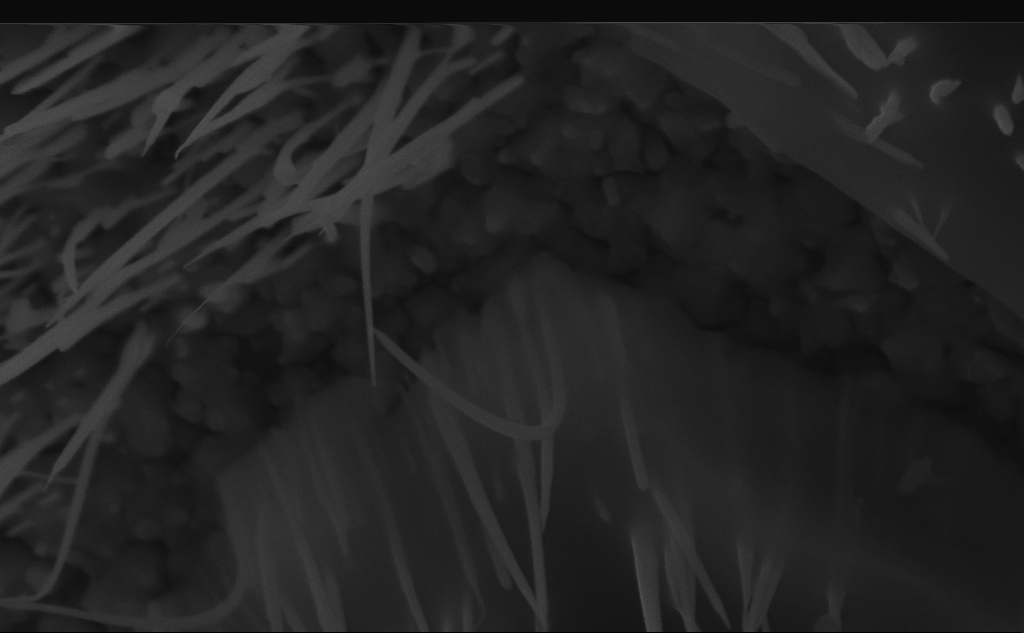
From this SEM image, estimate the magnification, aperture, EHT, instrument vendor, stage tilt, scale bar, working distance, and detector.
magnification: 98.96 K X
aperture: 30 µm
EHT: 10 kV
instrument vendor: Zeiss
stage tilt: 45°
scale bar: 200 nm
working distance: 6 mm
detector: InLens